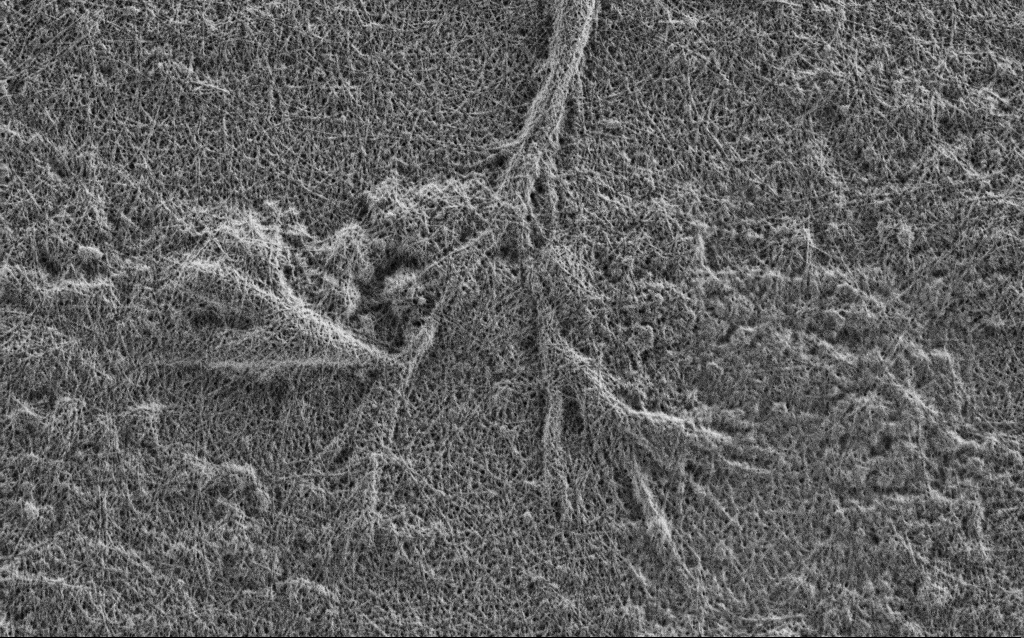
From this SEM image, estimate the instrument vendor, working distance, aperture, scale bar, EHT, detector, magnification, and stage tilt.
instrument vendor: Zeiss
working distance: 4 mm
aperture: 30 µm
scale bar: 2000 nm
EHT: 1 kV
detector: SE2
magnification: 15 K X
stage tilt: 0°